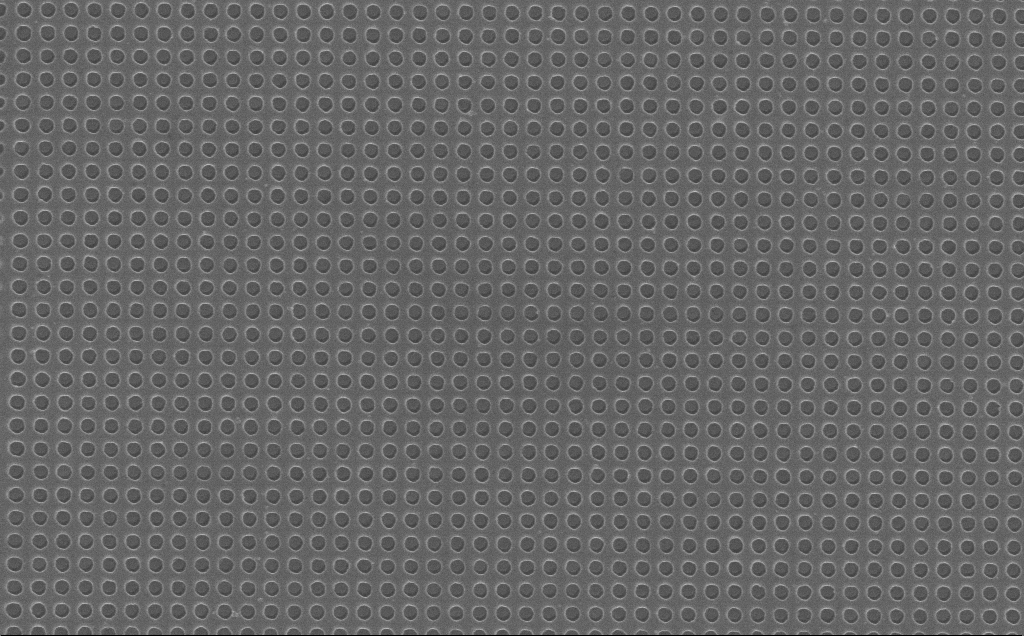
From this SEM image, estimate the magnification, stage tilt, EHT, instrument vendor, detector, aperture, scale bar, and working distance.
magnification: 34.2 K X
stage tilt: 0°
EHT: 10 kV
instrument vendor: Zeiss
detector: InLens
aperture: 30 µm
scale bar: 2000 nm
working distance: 7 mm